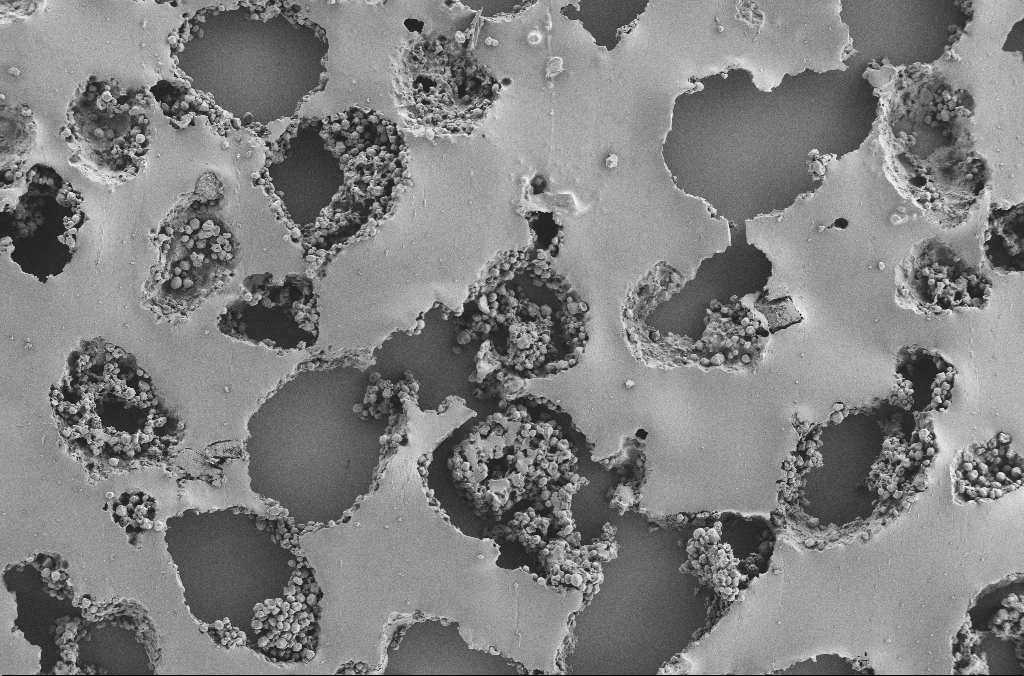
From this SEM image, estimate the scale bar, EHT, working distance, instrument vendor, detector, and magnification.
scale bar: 100000 nm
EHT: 2 kV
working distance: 3.7 mm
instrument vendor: Zeiss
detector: SE2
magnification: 0.25 K X